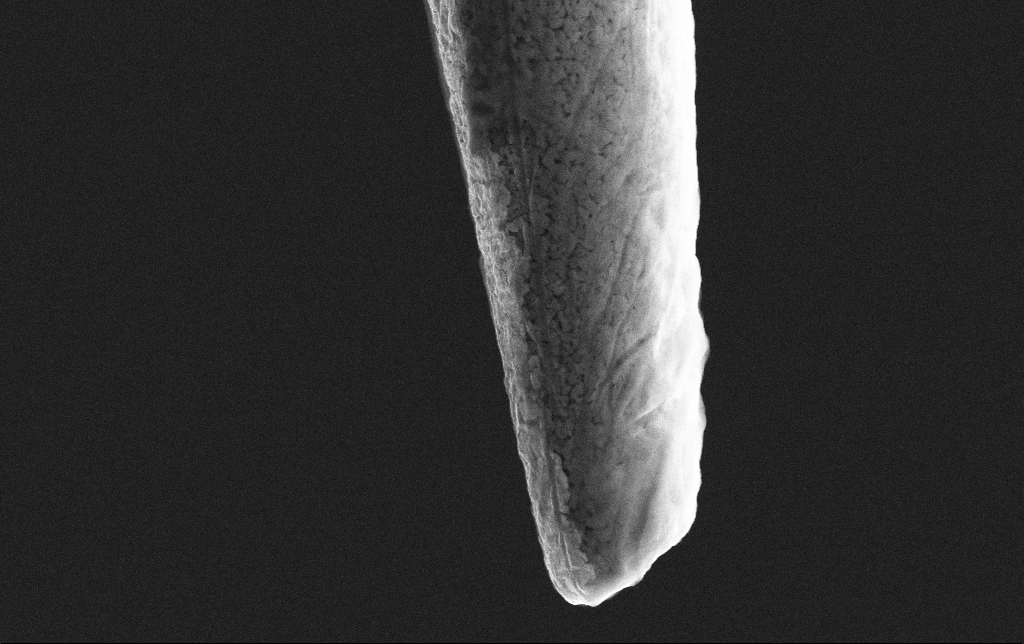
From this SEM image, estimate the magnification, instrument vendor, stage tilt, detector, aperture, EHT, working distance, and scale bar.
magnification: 42.25 K X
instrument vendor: Zeiss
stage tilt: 0°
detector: SE2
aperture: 30 µm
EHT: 10 kV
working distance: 8.2 mm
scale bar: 1000 nm